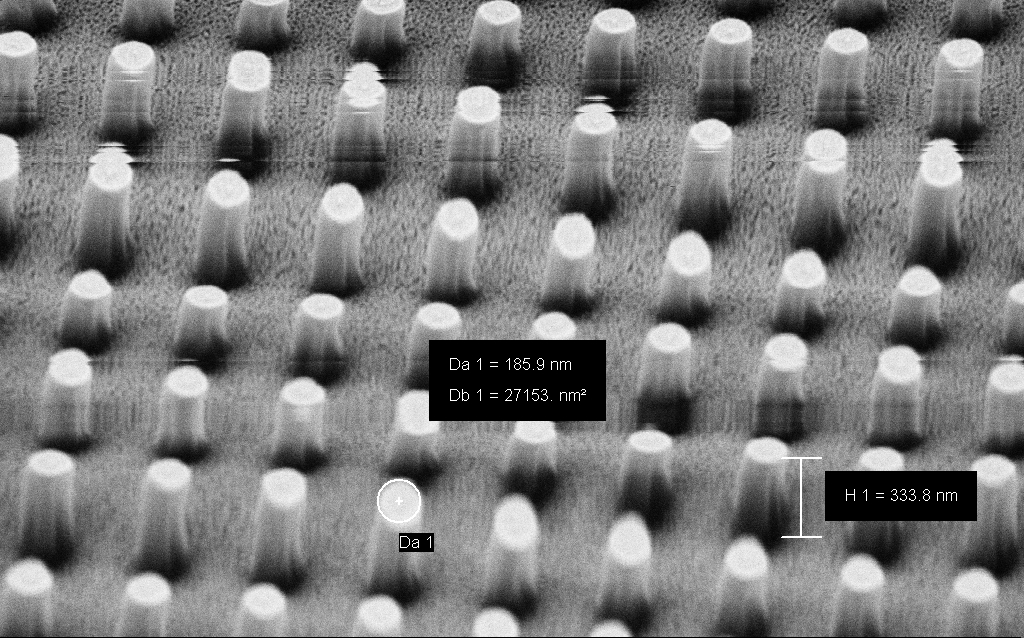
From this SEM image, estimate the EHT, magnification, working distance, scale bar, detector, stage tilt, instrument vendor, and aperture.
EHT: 3 kV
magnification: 86.89 K X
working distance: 5.7 mm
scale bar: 200 nm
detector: SE2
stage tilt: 45°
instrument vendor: Zeiss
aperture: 30 µm